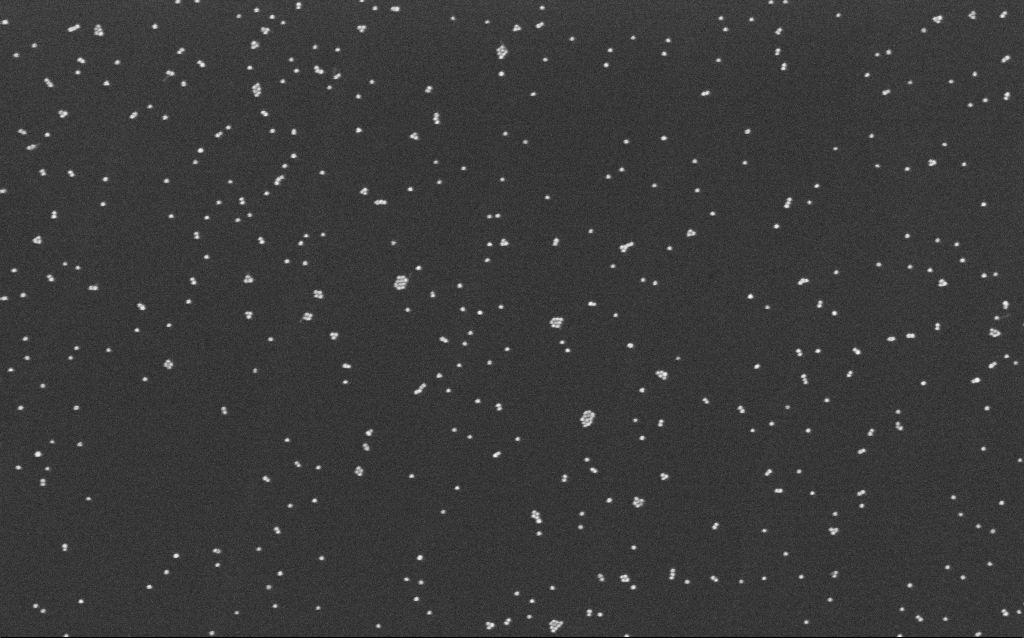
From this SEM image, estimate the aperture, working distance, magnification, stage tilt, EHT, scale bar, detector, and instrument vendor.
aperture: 30 µm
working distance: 6.5 mm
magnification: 100 K X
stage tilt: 0°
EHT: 10 kV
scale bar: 200 nm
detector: InLens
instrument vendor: Zeiss